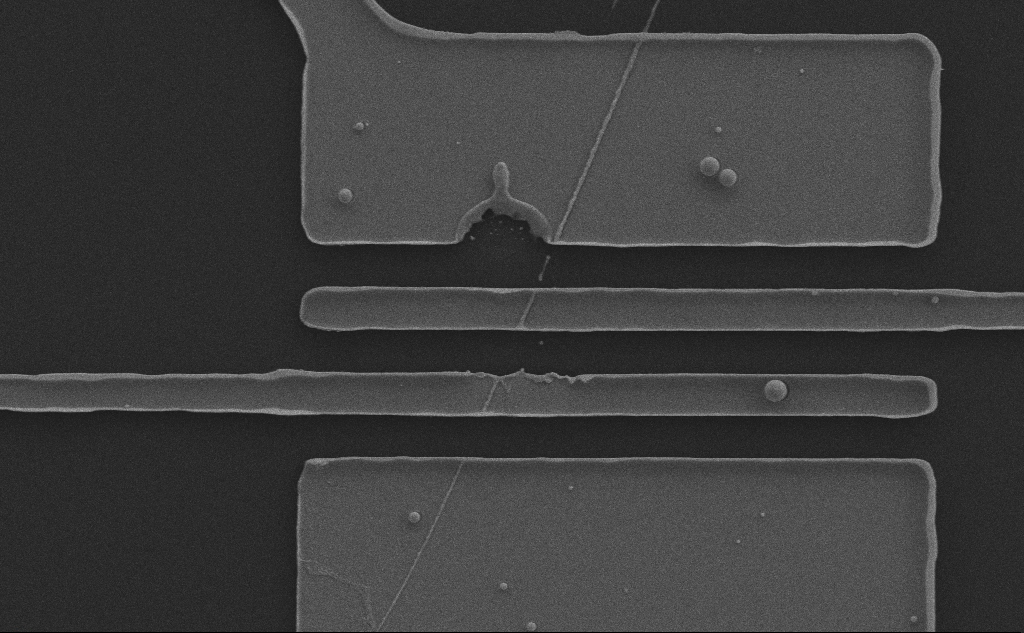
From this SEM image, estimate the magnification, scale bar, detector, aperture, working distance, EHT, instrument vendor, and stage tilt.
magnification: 7.89 K X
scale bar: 2000 nm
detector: SE2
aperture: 30 µm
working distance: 6 mm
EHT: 10 kV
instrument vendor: Zeiss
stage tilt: -0.1°